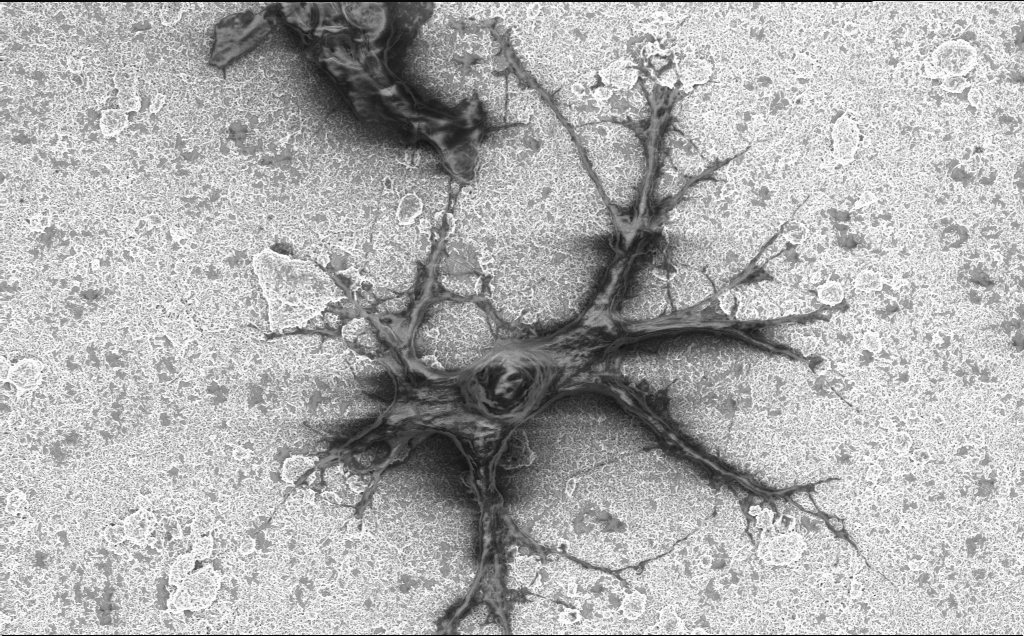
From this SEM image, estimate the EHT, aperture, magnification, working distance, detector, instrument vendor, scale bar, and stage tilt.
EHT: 2 kV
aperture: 30 µm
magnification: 5 K X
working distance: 7.1 mm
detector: InLens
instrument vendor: Zeiss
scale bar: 10000 nm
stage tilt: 0°